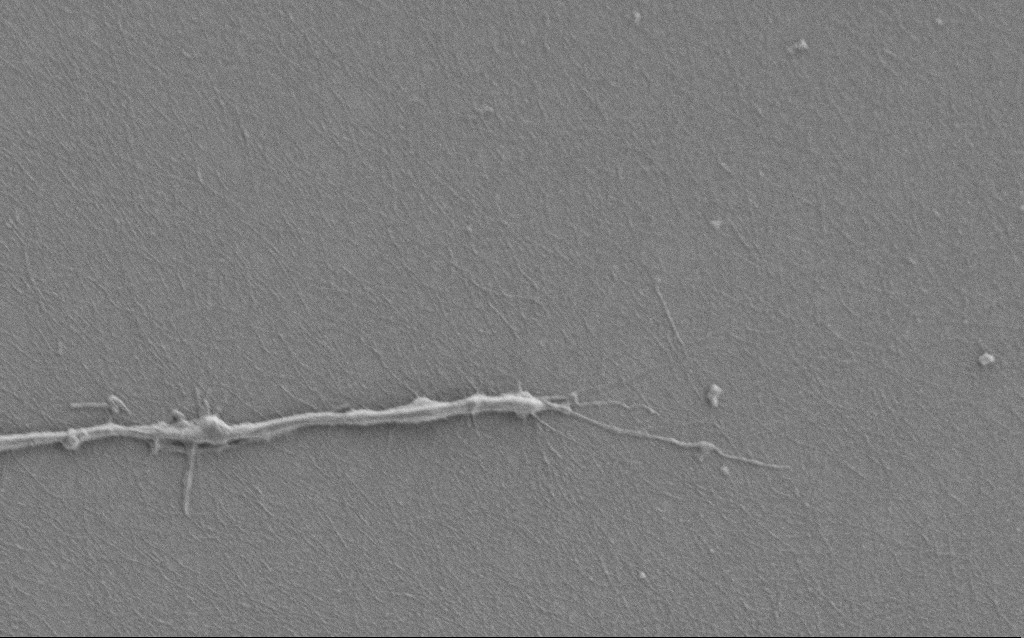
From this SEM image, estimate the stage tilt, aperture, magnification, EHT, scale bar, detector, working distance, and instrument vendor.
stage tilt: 0°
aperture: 30 µm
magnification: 7.5 K X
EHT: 1 kV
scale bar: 2000 nm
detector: SE2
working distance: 6 mm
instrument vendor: Zeiss